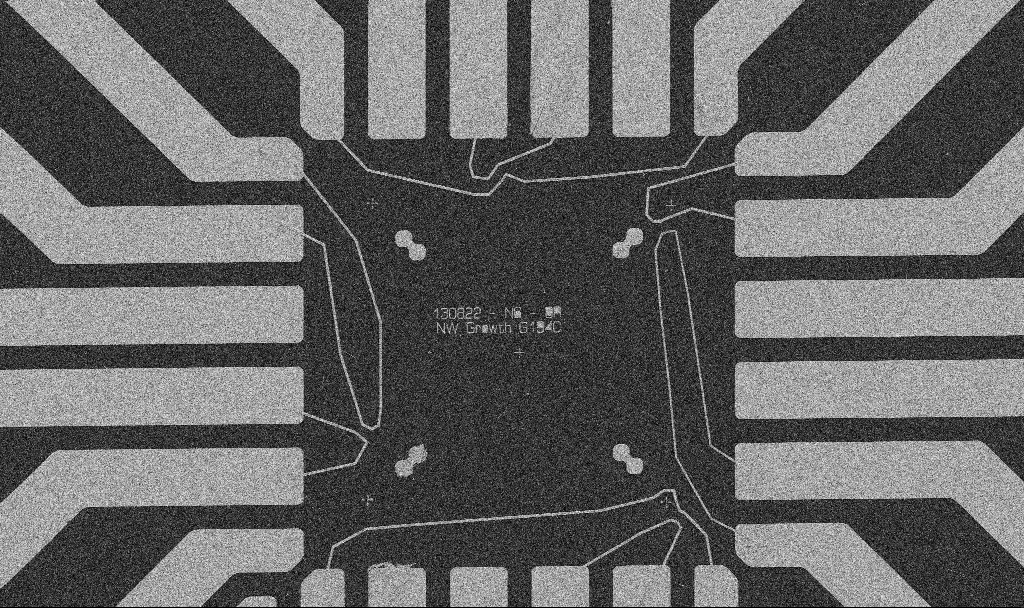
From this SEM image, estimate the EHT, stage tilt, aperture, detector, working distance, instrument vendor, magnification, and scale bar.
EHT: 5 kV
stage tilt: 0°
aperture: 30 µm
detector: SE2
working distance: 7.6 mm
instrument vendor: Zeiss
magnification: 1 K X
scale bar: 20000 nm